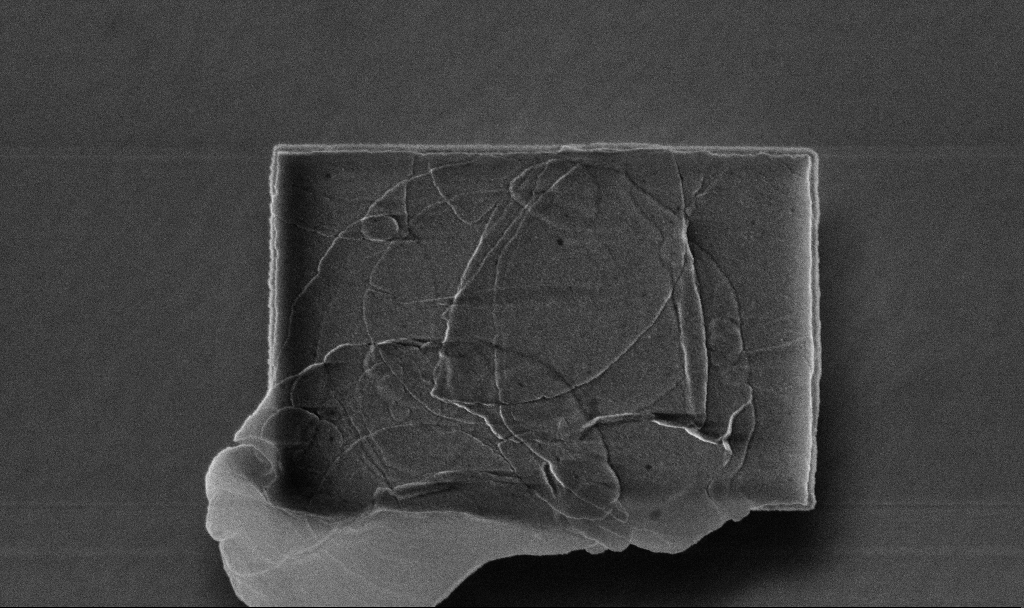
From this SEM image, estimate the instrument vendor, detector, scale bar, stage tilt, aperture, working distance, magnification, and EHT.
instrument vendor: Zeiss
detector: InLens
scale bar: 1000 nm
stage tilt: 0°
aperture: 30 µm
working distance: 3.2 mm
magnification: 44.17 K X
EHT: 5 kV